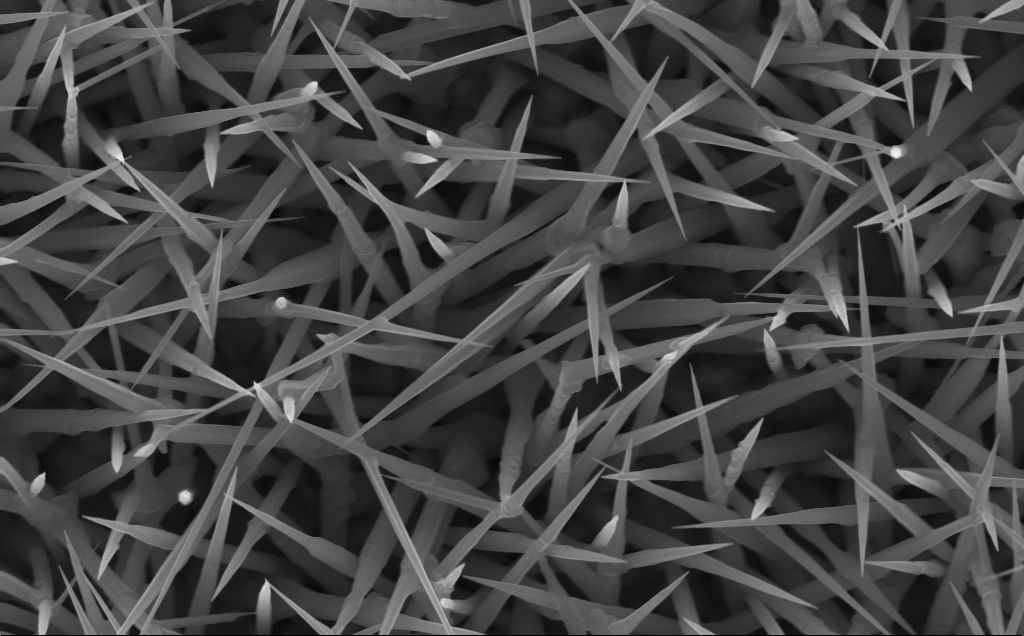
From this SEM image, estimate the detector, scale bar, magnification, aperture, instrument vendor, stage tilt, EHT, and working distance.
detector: InLens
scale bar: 1000 nm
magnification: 40 K X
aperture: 30 µm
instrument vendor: Zeiss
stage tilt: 0°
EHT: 10 kV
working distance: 4 mm